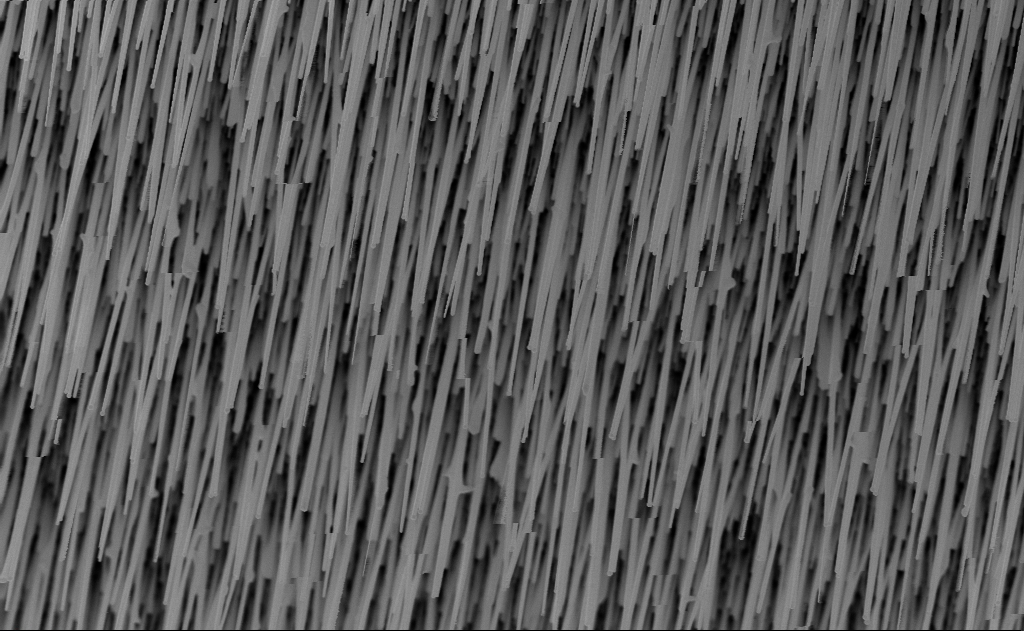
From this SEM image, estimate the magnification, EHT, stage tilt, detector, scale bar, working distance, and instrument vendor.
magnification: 20 K X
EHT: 10 kV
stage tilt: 0°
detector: InLens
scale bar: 2000 nm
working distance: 9 mm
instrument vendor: Zeiss